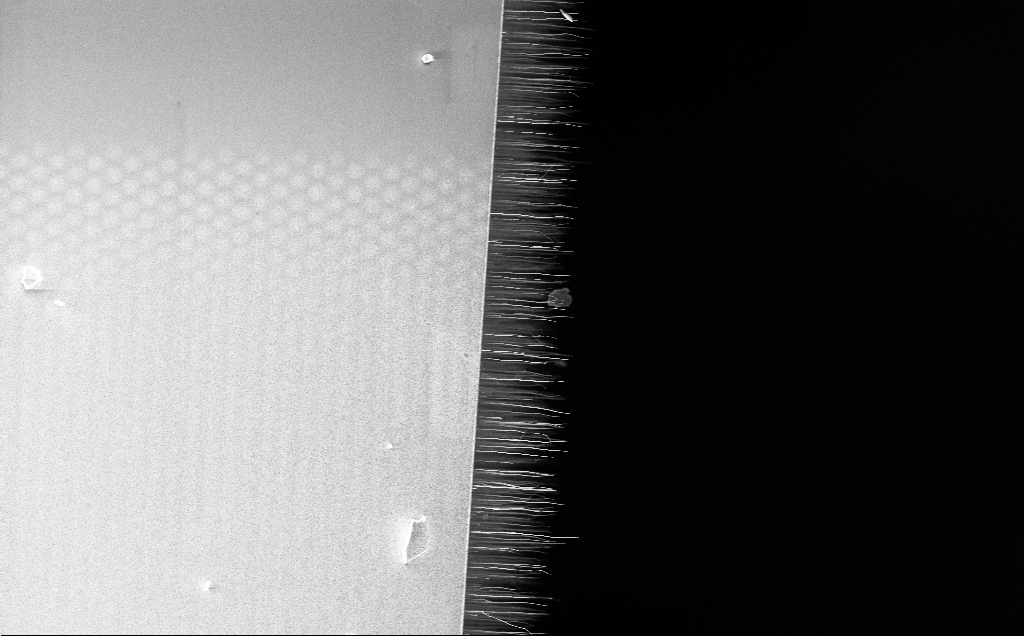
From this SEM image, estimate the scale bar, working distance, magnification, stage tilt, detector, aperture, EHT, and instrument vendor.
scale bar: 10000 nm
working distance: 12 mm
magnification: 2.6 K X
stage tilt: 0°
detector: InLens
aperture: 30 µm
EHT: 5 kV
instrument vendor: Zeiss